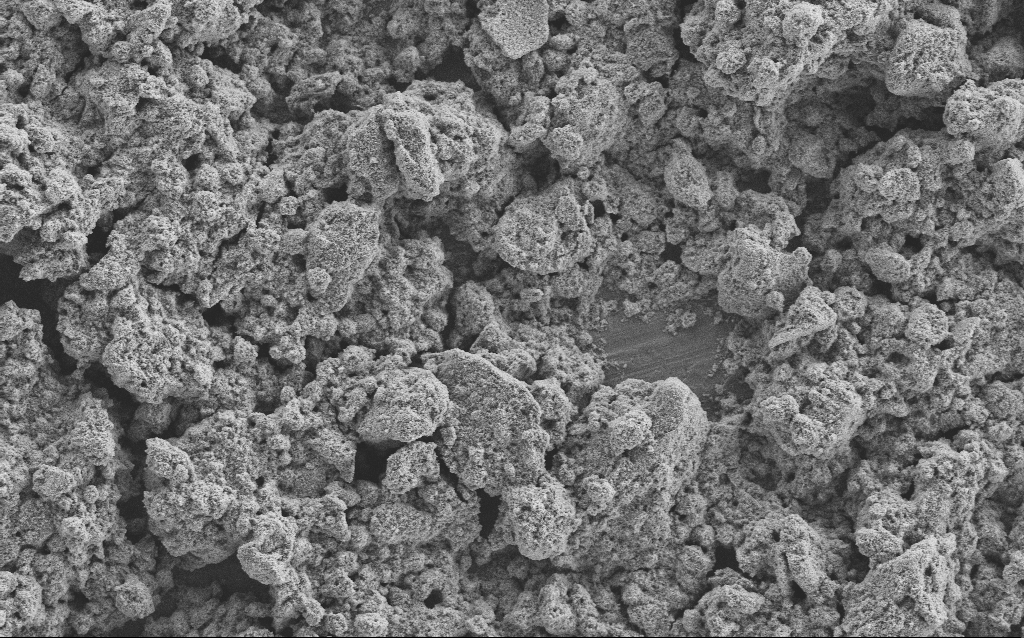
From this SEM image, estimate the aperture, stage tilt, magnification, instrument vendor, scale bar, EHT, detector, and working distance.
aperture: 30 µm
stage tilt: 0°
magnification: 1.23 K X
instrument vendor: Zeiss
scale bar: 10000 nm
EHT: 5 kV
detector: SE2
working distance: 4.1 mm